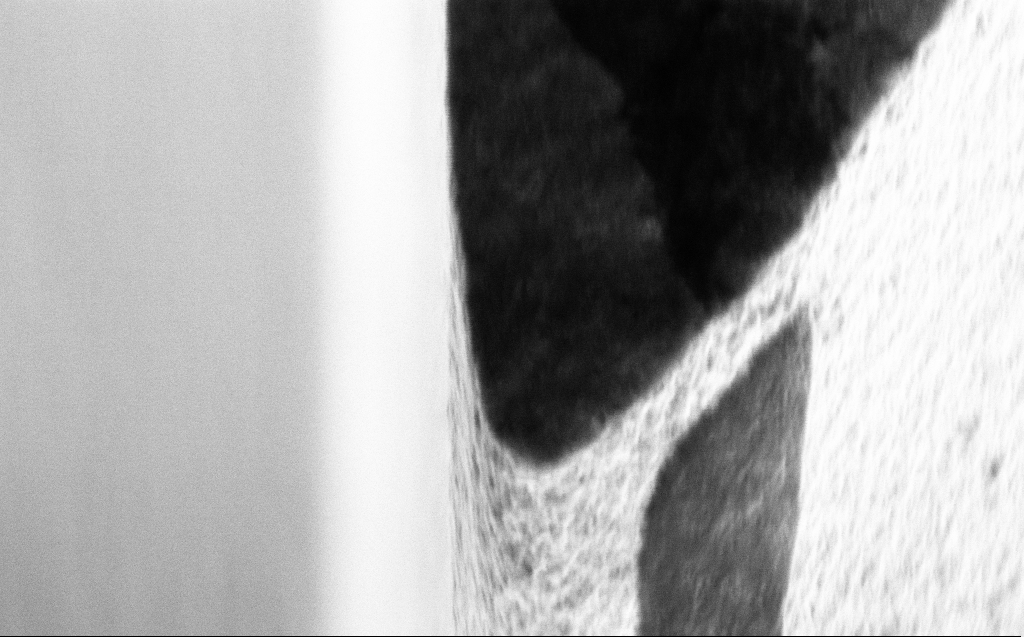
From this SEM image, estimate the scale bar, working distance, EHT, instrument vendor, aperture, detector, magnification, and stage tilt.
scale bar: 200 nm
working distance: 5 mm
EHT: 3 kV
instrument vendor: Zeiss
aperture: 30 µm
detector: InLens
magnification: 78.38 K X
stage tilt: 45°